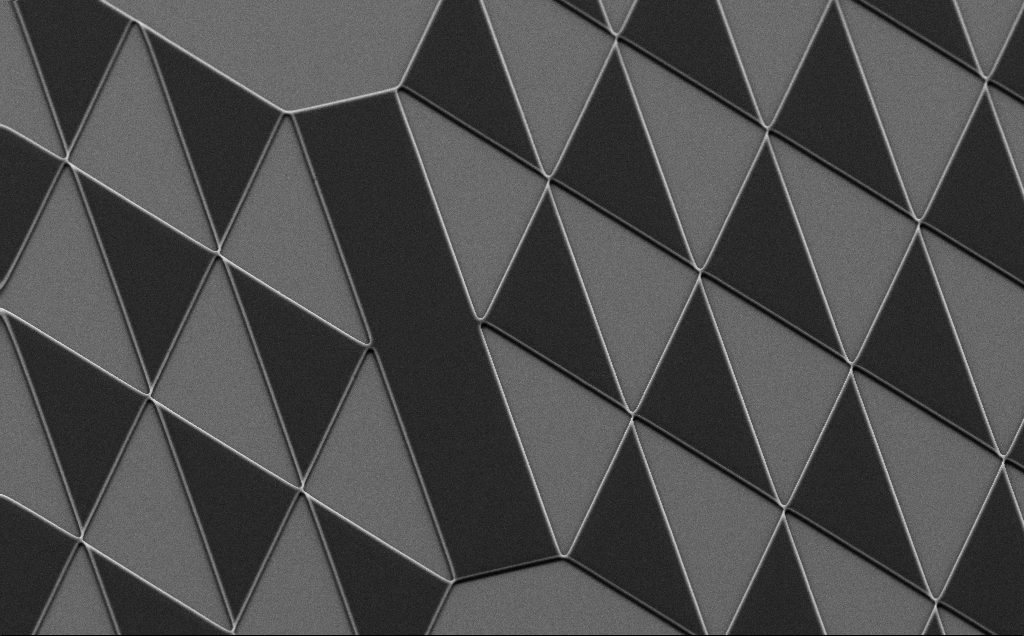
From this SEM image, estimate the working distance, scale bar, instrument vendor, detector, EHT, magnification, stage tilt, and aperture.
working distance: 8 mm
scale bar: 20000 nm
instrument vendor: Zeiss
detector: SE2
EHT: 10 kV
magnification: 1.19 K X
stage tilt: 35°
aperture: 30 µm